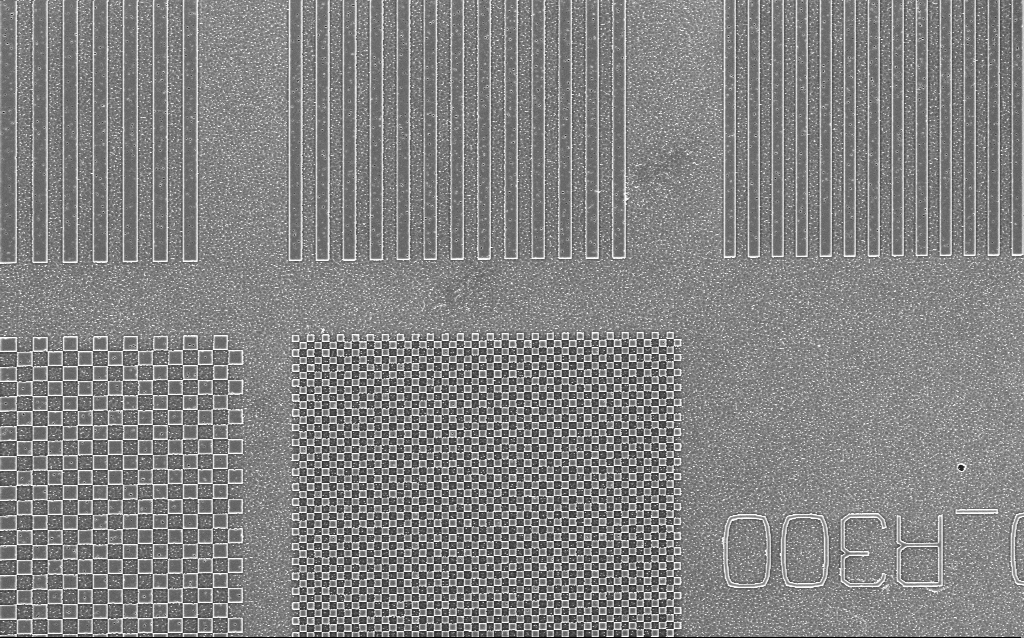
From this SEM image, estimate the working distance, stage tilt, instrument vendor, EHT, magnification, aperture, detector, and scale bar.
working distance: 8 mm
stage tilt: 0°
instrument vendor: Zeiss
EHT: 3 kV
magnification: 5.52 K X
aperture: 30 µm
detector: InLens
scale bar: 10000 nm